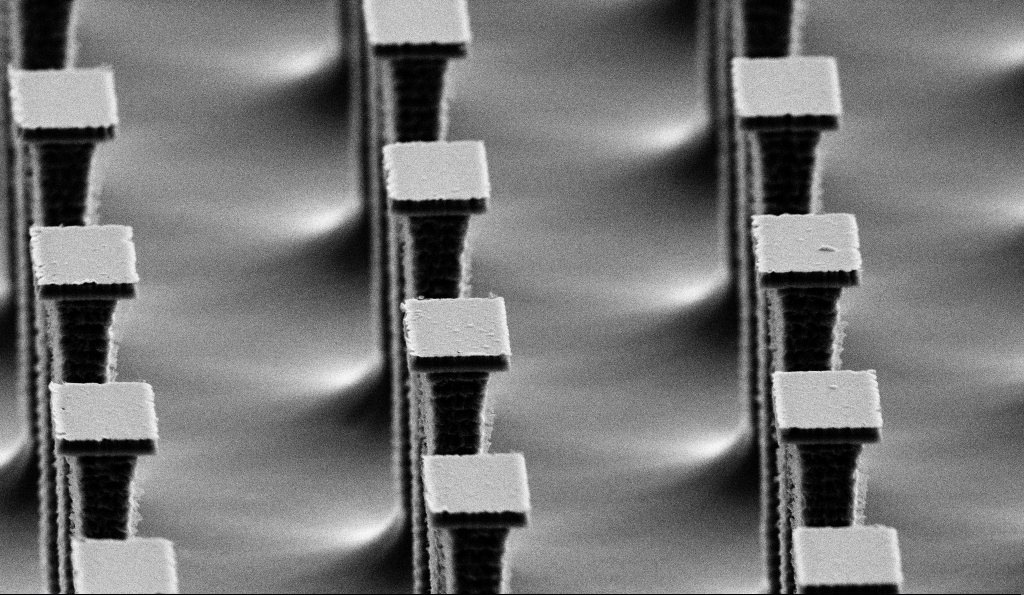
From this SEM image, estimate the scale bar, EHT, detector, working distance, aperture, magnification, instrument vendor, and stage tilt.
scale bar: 2000 nm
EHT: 5 kV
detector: SE2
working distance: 10.6 mm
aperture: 30 µm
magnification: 12.61 K X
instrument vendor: Zeiss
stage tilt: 70°